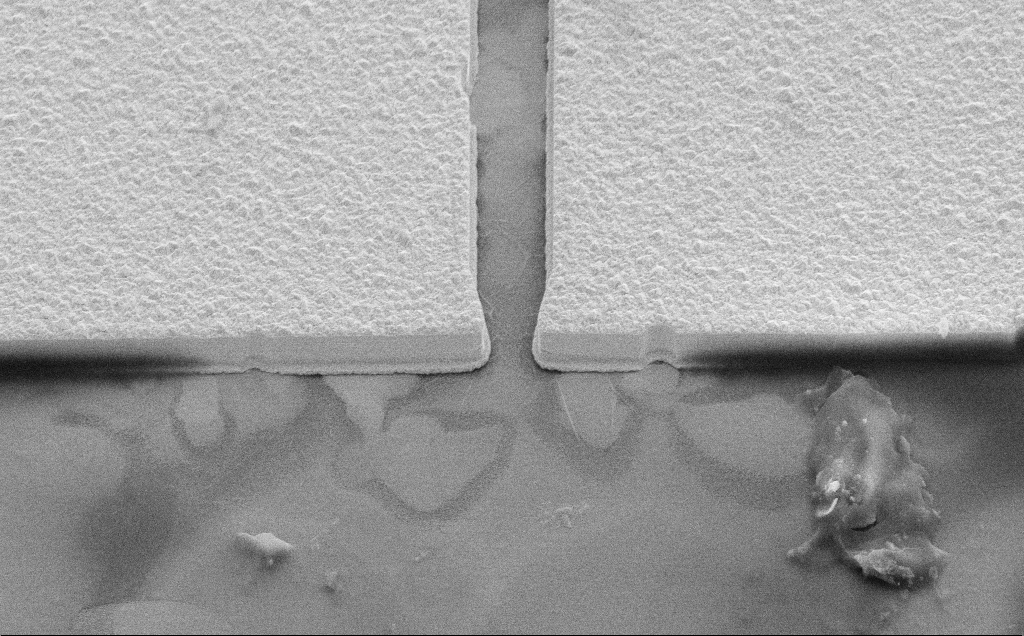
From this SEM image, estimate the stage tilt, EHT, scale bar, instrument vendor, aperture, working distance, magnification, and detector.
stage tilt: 45°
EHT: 10 kV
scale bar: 20000 nm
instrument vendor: Zeiss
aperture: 30 µm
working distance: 11 mm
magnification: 3.13 K X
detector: SE2